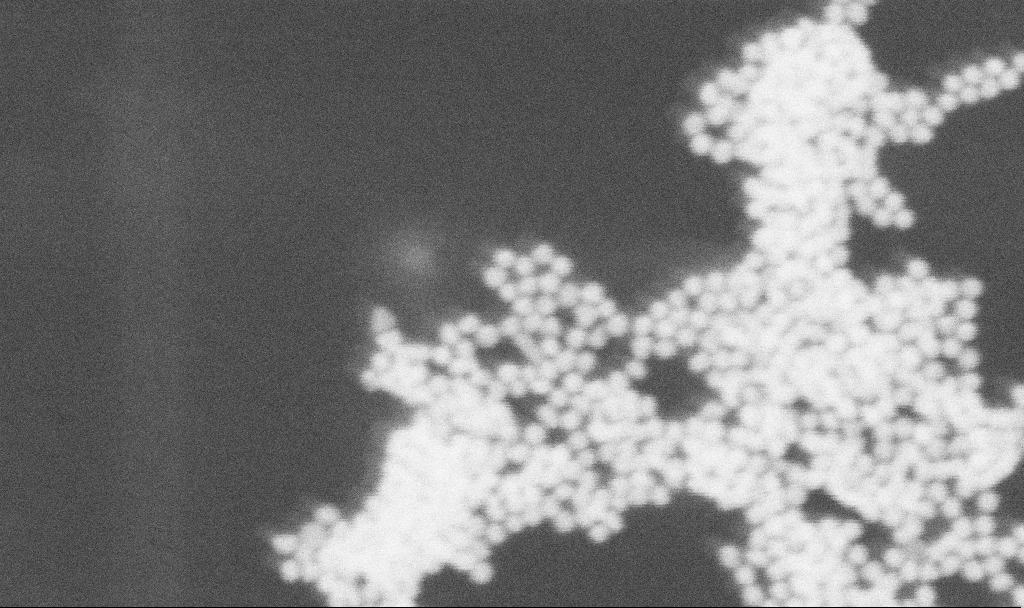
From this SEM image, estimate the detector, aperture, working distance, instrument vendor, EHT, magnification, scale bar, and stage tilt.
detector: SE2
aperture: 30 µm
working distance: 11.3 mm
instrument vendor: Zeiss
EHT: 25 kV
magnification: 400 K X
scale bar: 100 nm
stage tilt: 0°